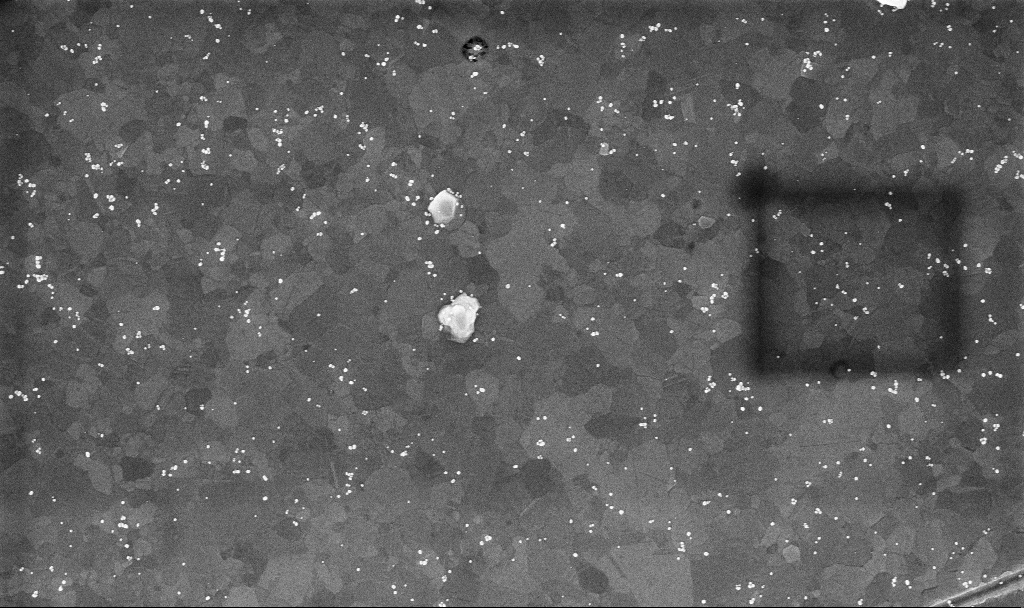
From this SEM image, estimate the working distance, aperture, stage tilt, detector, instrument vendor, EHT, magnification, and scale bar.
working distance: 3.4 mm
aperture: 30 µm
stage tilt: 0°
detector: InLens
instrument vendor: Zeiss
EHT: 10 kV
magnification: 50.84 K X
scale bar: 1000 nm